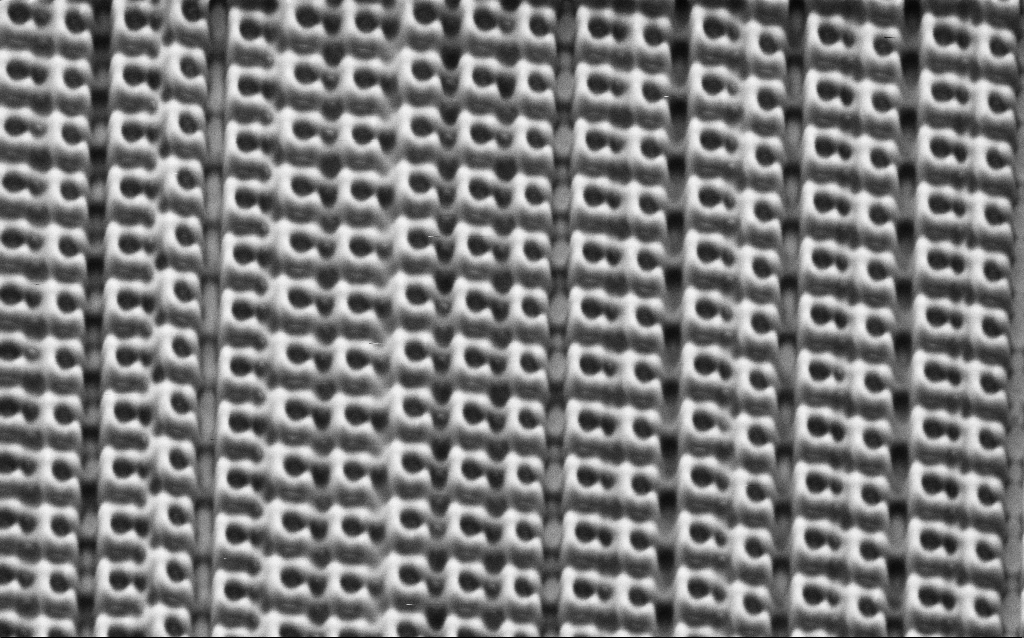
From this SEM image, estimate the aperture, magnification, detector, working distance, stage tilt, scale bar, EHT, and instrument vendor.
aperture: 30 µm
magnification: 43.42 K X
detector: SE2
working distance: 7.2 mm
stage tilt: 30°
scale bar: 1000 nm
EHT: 1.5 kV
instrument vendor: Zeiss